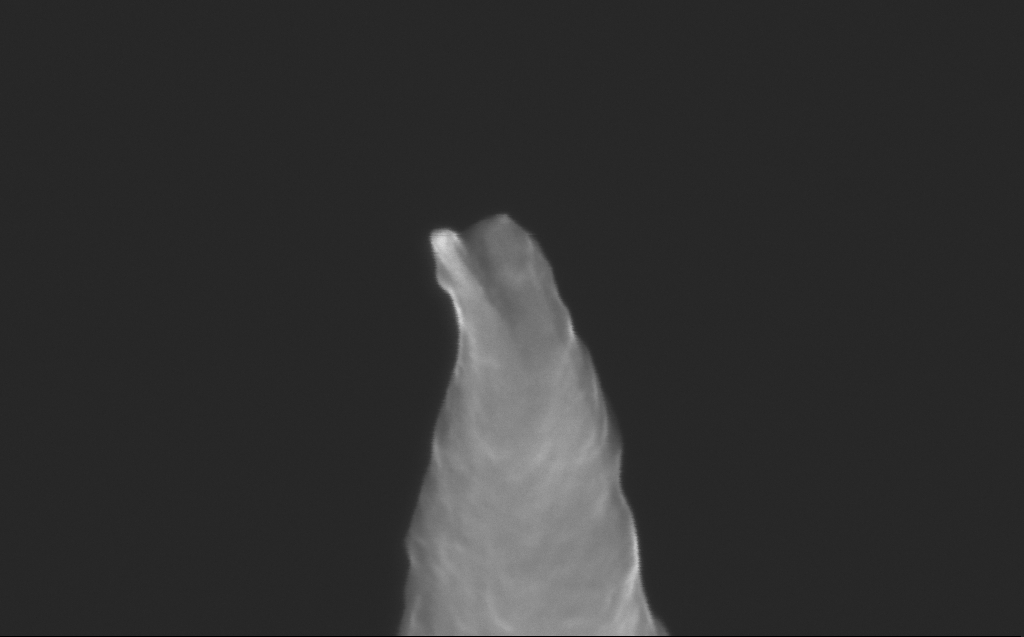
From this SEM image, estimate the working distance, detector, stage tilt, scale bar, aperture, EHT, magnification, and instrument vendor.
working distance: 4 mm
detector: InLens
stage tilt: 40°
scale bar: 200 nm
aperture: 30 µm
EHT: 10 kV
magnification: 312.82 K X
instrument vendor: Zeiss